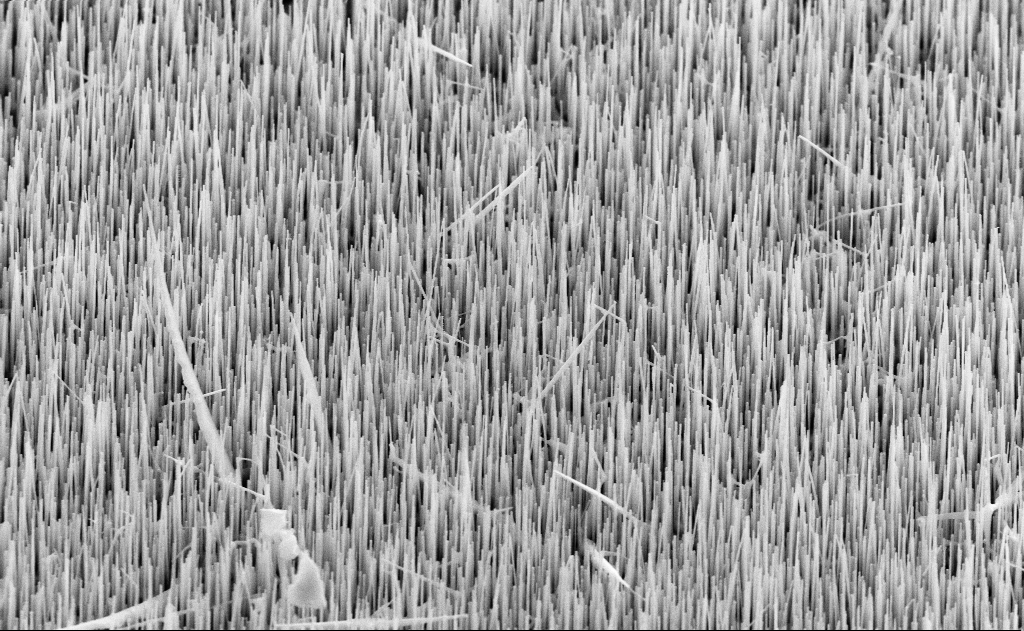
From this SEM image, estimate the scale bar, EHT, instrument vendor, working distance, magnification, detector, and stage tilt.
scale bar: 1000 nm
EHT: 10 kV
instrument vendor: Zeiss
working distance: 11 mm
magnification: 20 K X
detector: SE2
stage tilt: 45°